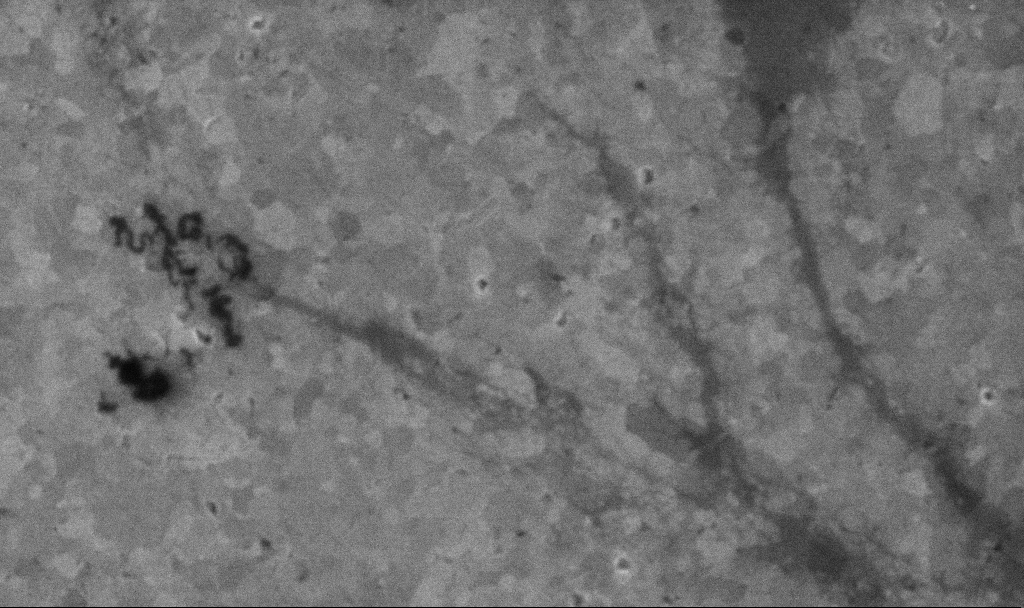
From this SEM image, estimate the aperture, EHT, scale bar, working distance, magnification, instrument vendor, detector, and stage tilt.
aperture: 30 µm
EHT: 10 kV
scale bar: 1000 nm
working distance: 3.1 mm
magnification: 50.56 K X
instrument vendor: Zeiss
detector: InLens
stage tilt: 0°